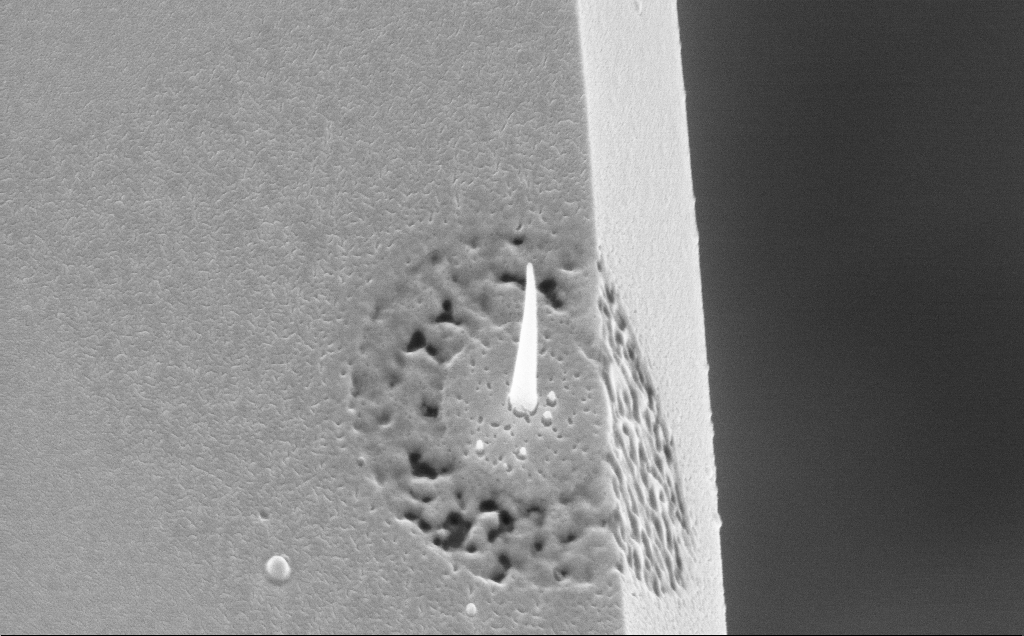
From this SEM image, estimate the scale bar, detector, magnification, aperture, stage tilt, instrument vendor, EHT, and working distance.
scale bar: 2000 nm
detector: InLens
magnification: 32.22 K X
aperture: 30 µm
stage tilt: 44.2°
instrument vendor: Zeiss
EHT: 10 kV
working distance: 3 mm